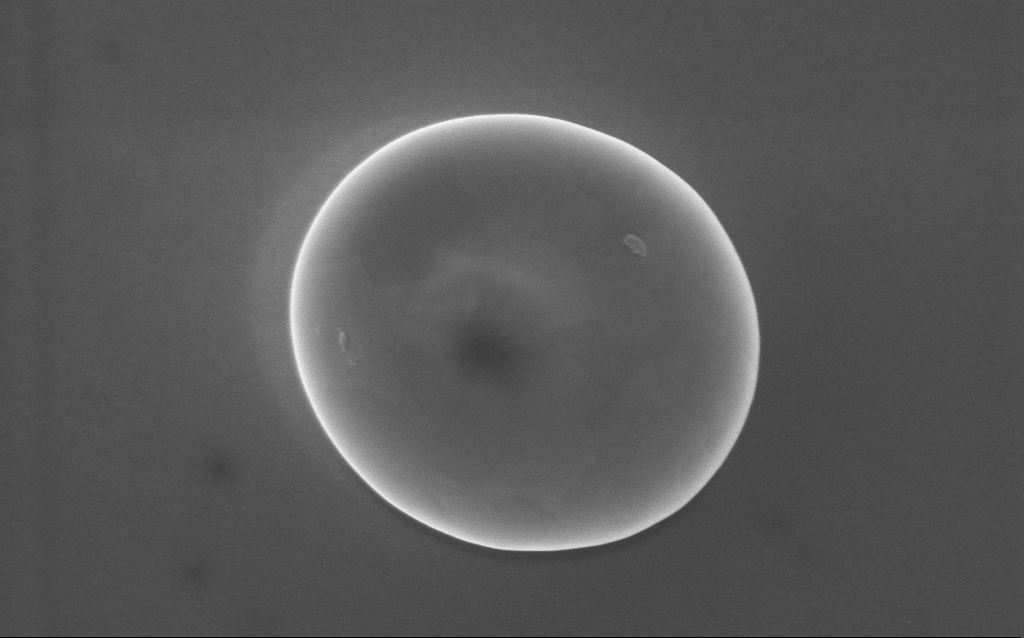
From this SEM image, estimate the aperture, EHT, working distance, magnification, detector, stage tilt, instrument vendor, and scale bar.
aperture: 30 µm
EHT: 5 kV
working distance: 3 mm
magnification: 100 K X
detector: InLens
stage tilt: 0°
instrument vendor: Zeiss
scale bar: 200 nm